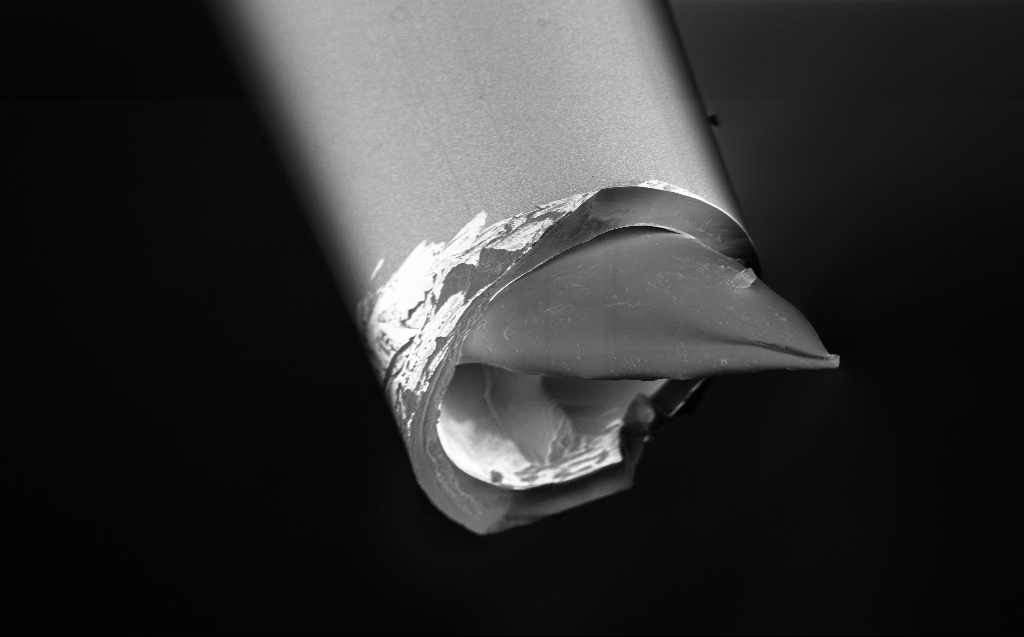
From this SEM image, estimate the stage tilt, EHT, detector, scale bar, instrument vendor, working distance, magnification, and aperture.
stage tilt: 45°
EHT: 1.5 kV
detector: InLens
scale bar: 20000 nm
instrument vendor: Zeiss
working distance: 3 mm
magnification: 1.89 K X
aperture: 30 µm